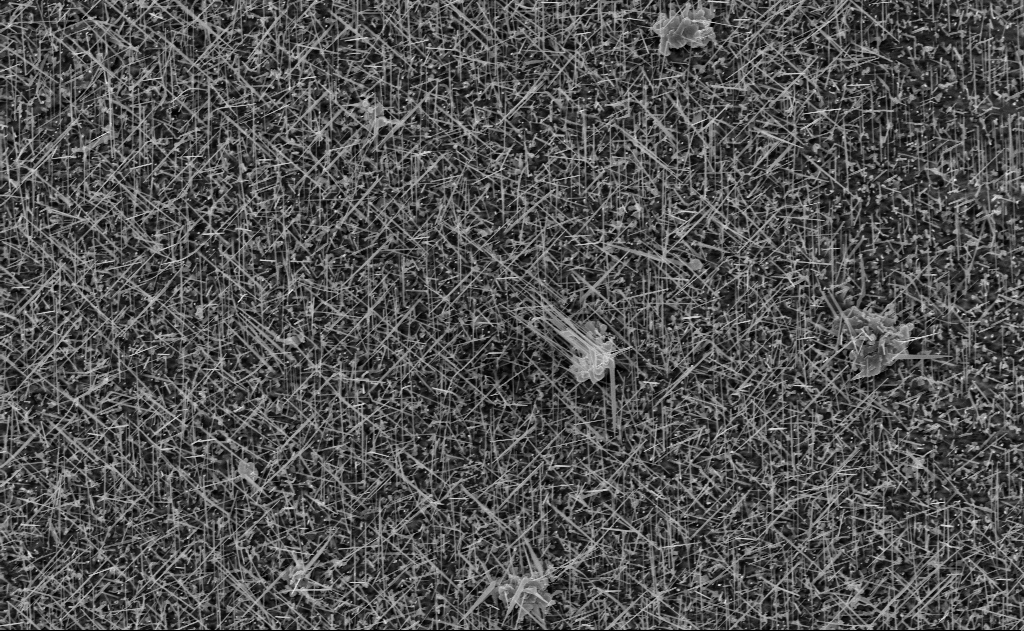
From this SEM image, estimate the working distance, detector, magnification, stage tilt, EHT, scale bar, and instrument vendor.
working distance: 15 mm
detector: InLens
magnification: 10 K X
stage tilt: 0°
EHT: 10 kV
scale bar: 2000 nm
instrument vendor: Zeiss